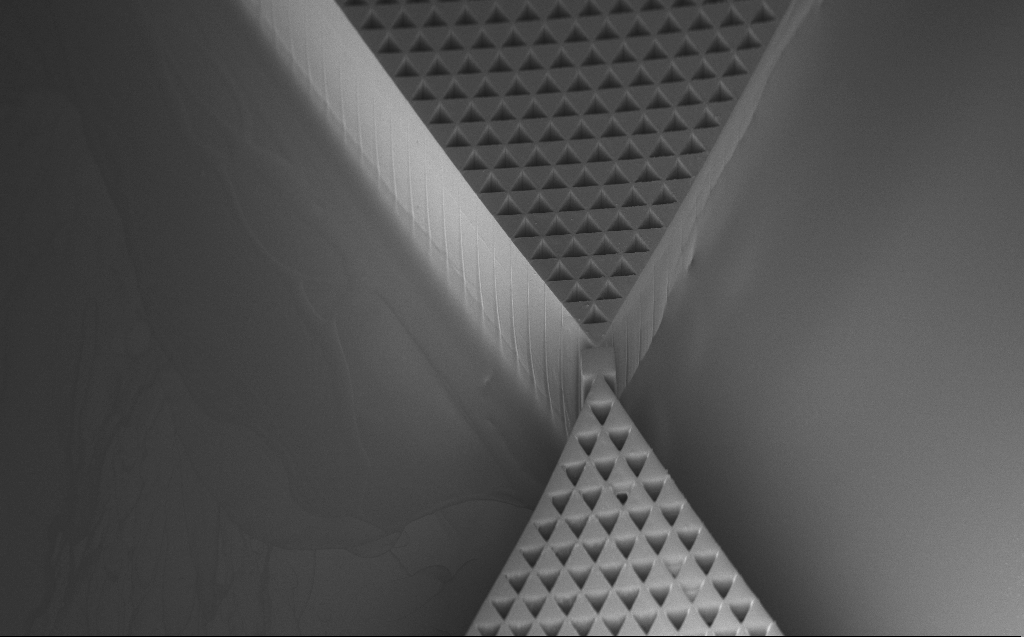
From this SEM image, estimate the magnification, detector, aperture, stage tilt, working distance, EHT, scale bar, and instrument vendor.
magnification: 0.193 K X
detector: InLens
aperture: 30 µm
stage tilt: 45°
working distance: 6 mm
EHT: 3 kV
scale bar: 200000 nm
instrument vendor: Zeiss